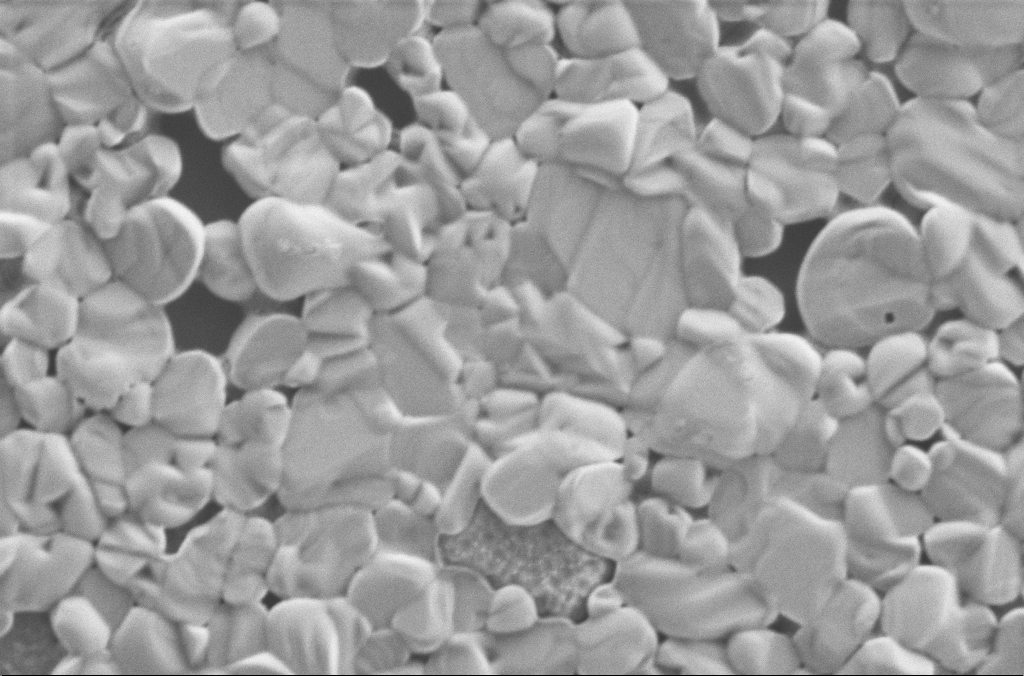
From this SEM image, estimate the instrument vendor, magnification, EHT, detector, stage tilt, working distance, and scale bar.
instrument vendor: Zeiss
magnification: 50 K X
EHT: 2 kV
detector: SE2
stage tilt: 0°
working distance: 3 mm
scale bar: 1000 nm